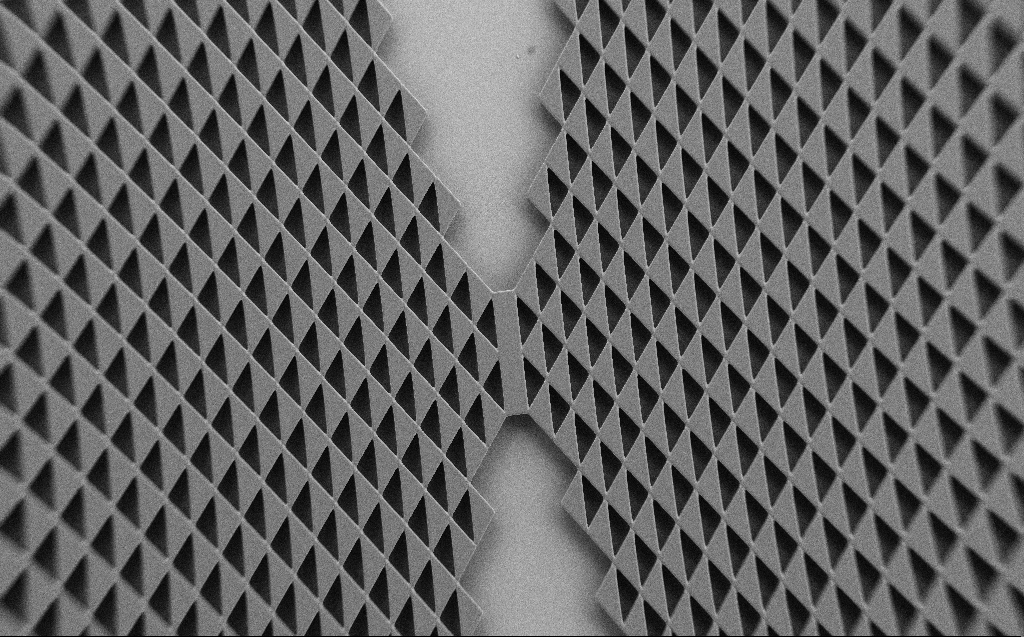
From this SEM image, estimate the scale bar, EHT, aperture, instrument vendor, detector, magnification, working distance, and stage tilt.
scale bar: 100000 nm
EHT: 1 kV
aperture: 30 µm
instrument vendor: Zeiss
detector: SE2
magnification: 0.228 K X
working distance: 6 mm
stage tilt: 0°